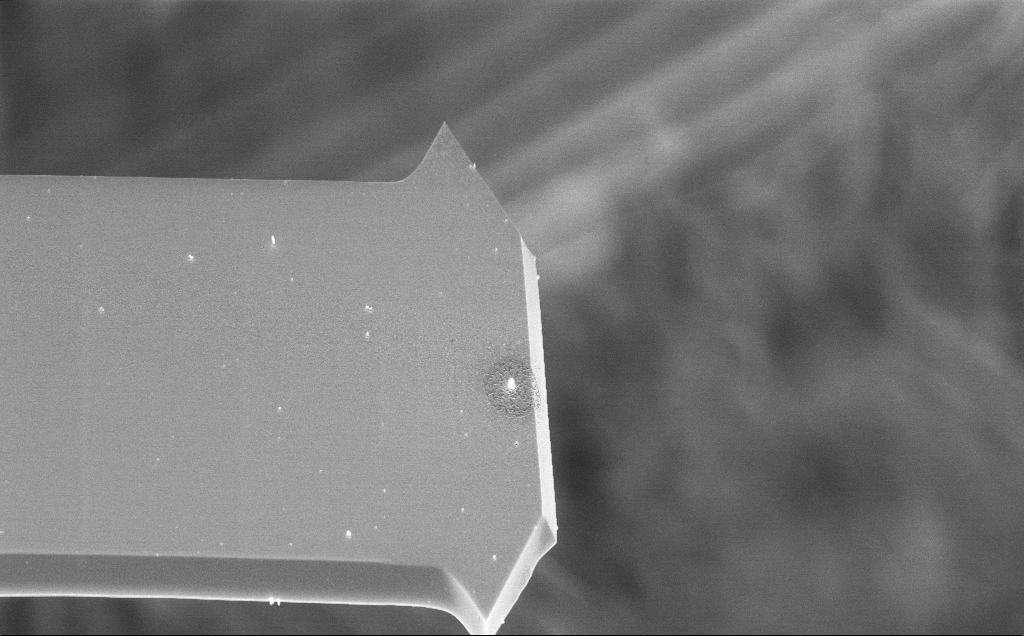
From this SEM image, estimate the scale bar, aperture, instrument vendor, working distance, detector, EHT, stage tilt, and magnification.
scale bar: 10000 nm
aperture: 30 µm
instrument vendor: Zeiss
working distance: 3 mm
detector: InLens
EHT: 10 kV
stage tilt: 44.2°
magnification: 4.23 K X